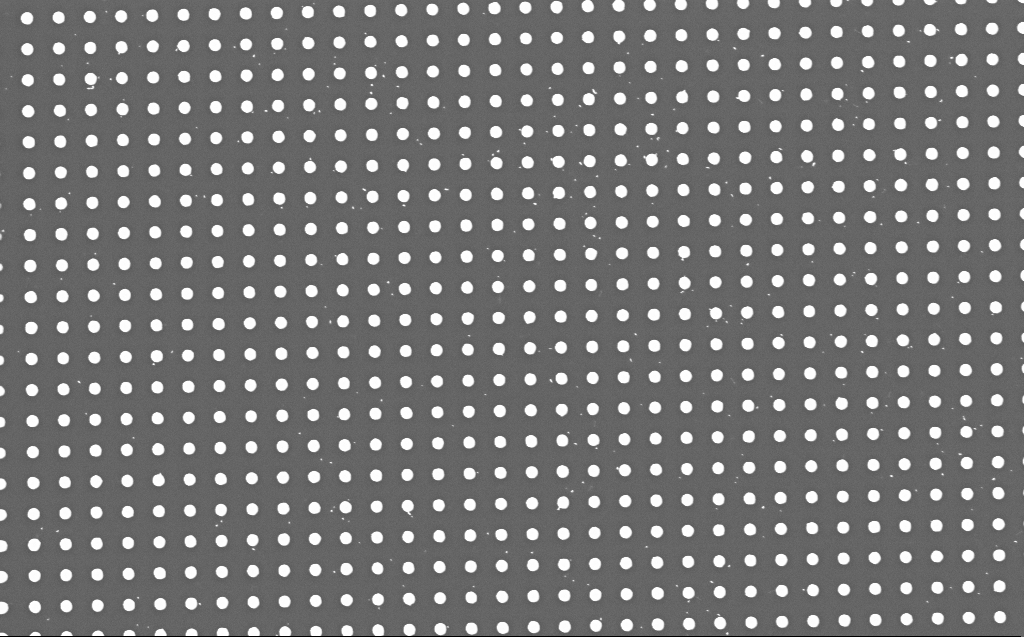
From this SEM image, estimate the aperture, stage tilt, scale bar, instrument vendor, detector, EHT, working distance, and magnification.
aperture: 30 µm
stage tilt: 0°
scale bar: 2000 nm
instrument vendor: Zeiss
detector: InLens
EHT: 5 kV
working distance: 5 mm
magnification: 10.94 K X